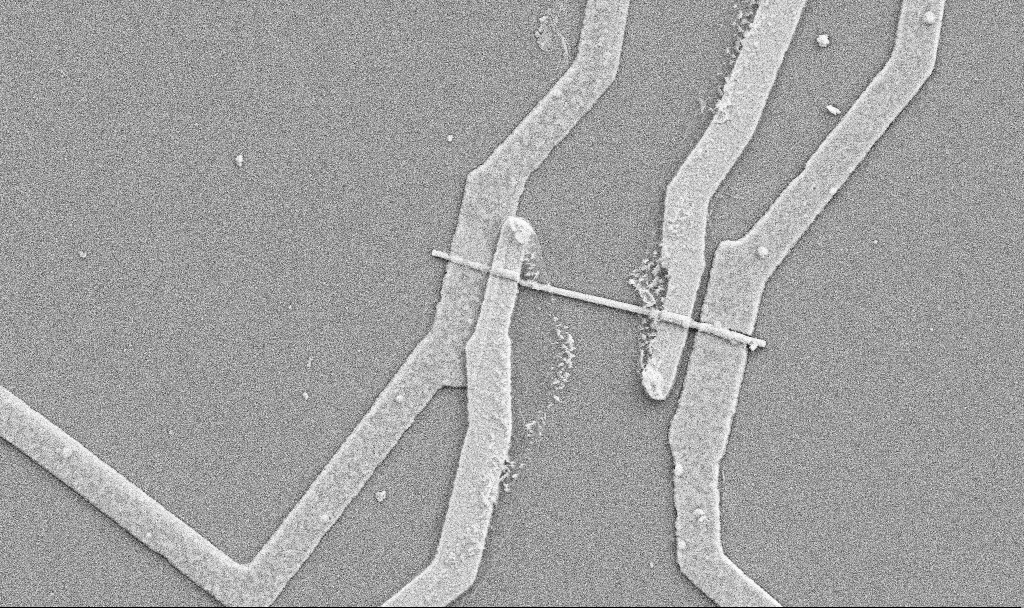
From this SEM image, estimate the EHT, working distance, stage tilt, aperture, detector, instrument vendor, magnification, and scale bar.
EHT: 5 kV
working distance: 10.7 mm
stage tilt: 0°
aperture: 30 µm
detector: SE2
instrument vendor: Zeiss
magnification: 20 K X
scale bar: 1000 nm